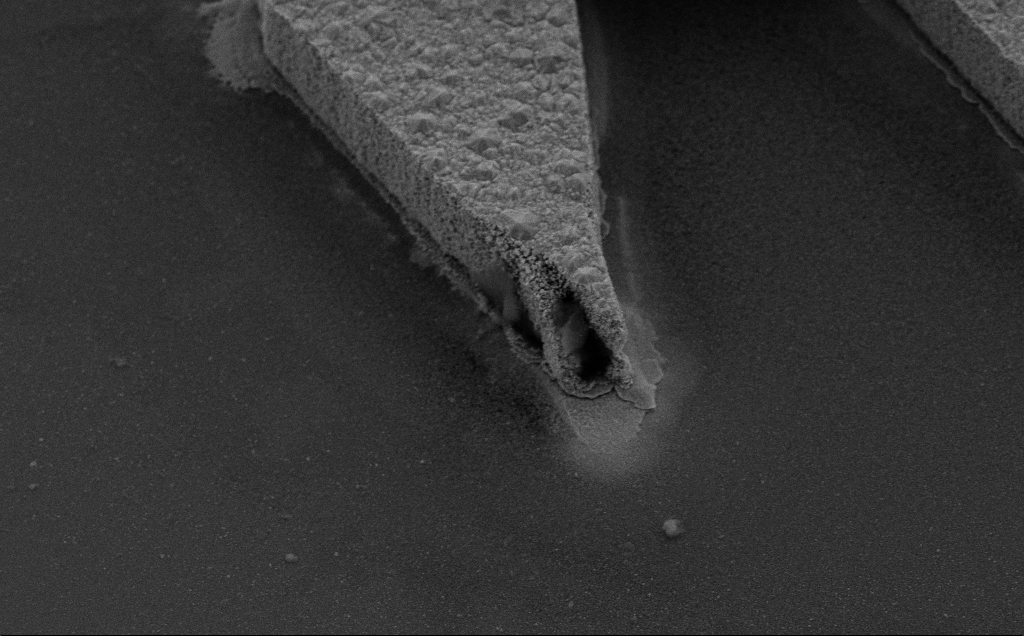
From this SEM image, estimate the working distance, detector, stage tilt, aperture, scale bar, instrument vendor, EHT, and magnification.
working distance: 8 mm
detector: SE2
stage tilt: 35°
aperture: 30 µm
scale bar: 2000 nm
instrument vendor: Zeiss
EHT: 10 kV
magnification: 9.41 K X